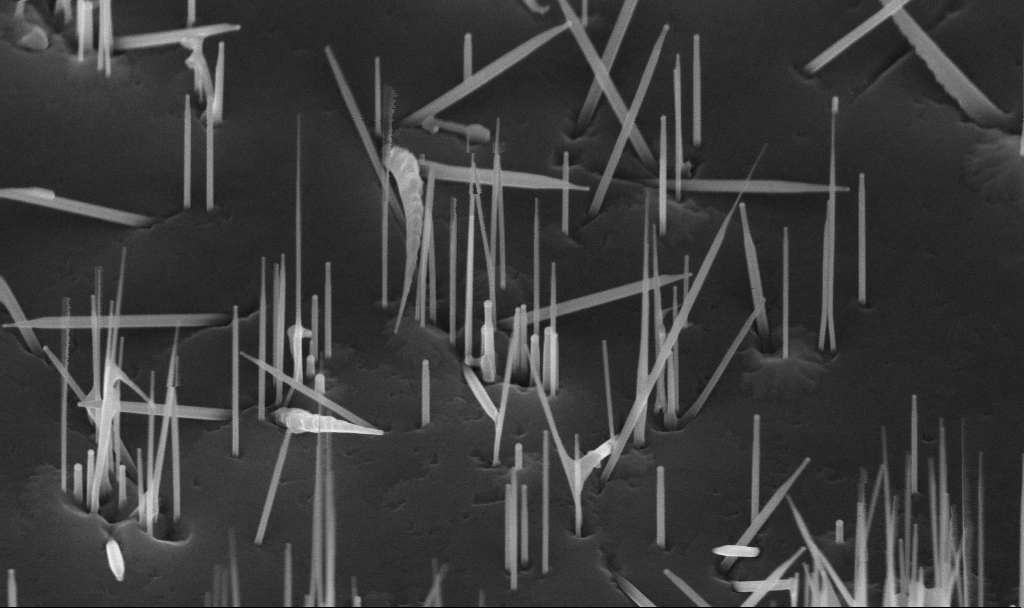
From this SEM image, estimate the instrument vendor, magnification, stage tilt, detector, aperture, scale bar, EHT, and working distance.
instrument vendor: Zeiss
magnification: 76.31 K X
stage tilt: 45°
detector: InLens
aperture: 30 µm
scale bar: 200 nm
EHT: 10 kV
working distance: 5.6 mm